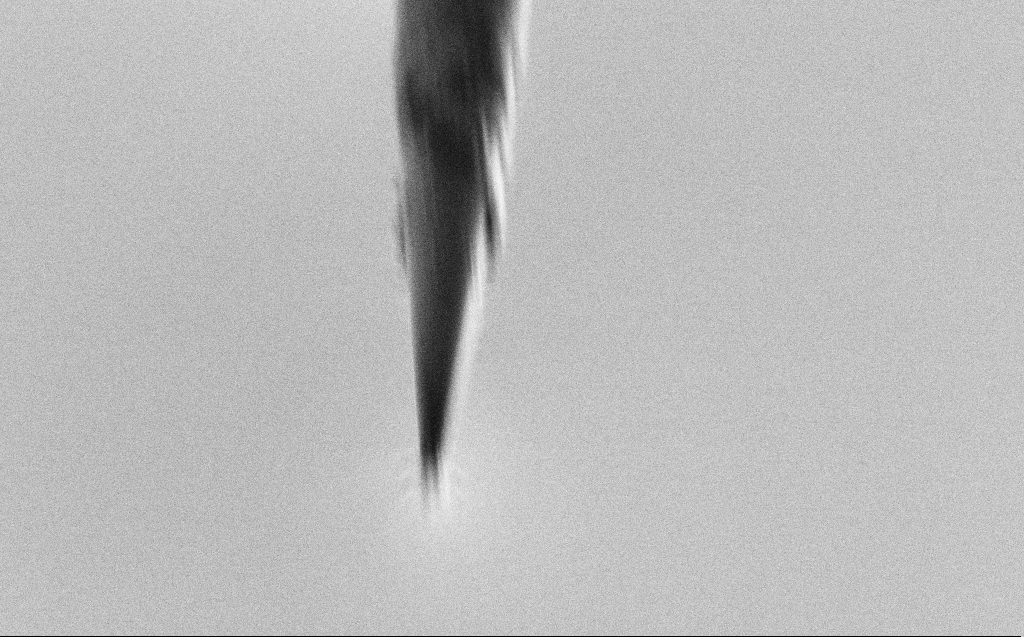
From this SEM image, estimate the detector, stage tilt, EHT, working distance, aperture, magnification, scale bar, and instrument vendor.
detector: SE2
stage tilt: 45°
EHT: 2 kV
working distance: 4 mm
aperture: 20 µm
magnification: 22.85 K X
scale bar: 2000 nm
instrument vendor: Zeiss